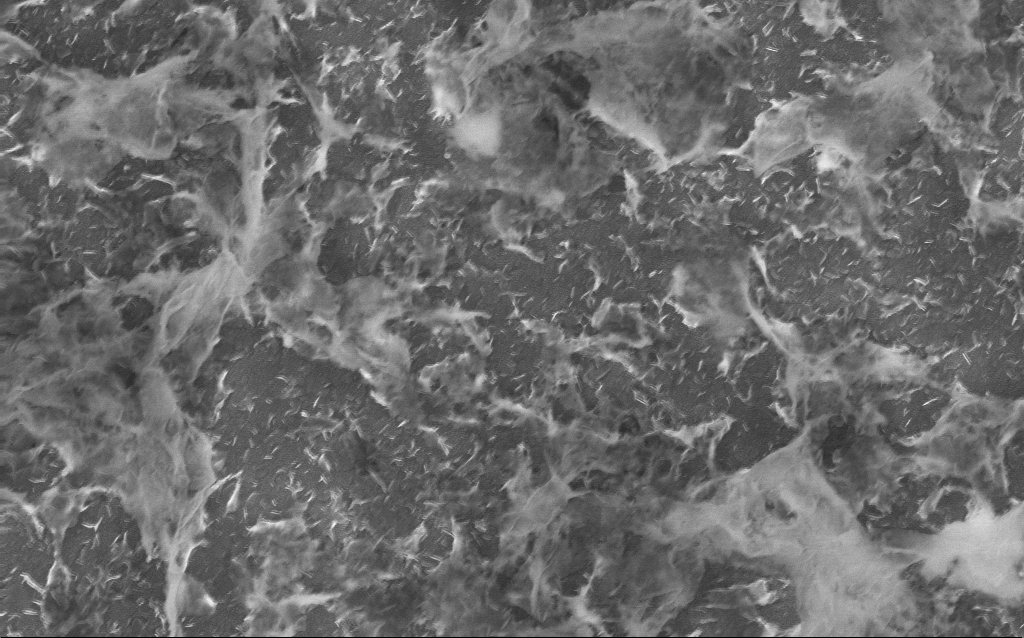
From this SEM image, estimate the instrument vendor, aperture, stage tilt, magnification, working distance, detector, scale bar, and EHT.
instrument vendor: Zeiss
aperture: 30 µm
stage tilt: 0°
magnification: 40 K X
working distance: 2.5 mm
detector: InLens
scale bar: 1000 nm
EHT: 10 kV